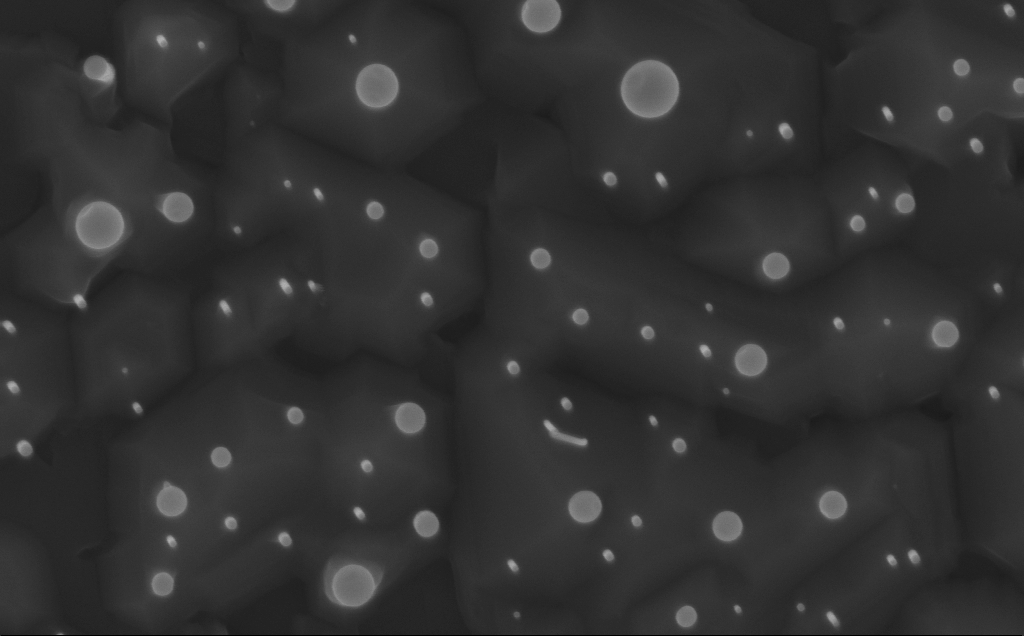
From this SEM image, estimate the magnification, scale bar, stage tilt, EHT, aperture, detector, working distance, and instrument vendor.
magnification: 80 K X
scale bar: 200 nm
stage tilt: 0°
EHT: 10 kV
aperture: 30 µm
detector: InLens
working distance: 3 mm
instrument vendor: Zeiss